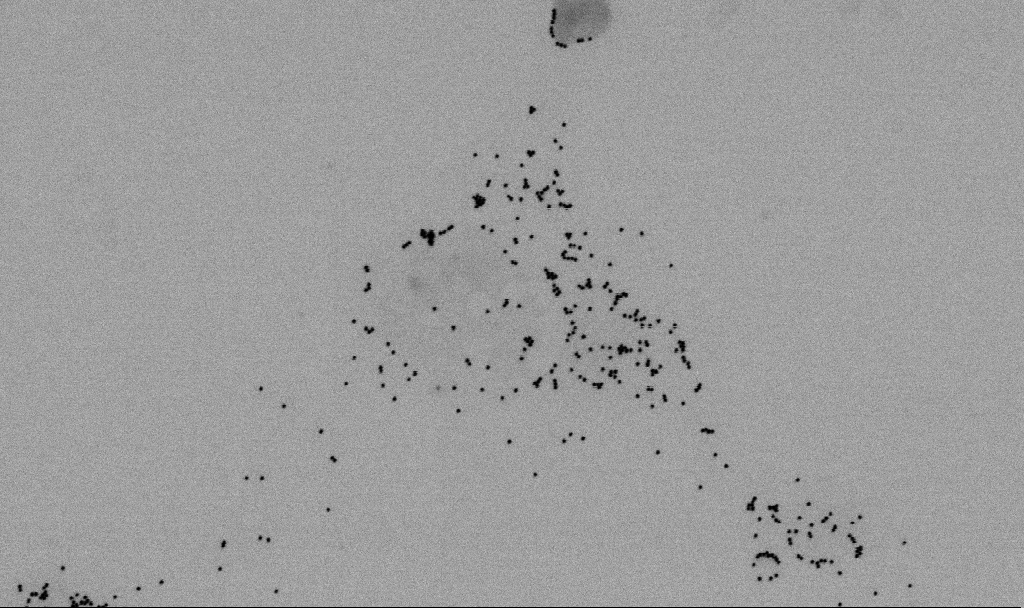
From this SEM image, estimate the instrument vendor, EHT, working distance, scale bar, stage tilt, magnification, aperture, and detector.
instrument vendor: Zeiss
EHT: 2 kV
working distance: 6.5 mm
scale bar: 1000 nm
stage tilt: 0°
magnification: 60 K X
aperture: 30 µm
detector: SE2